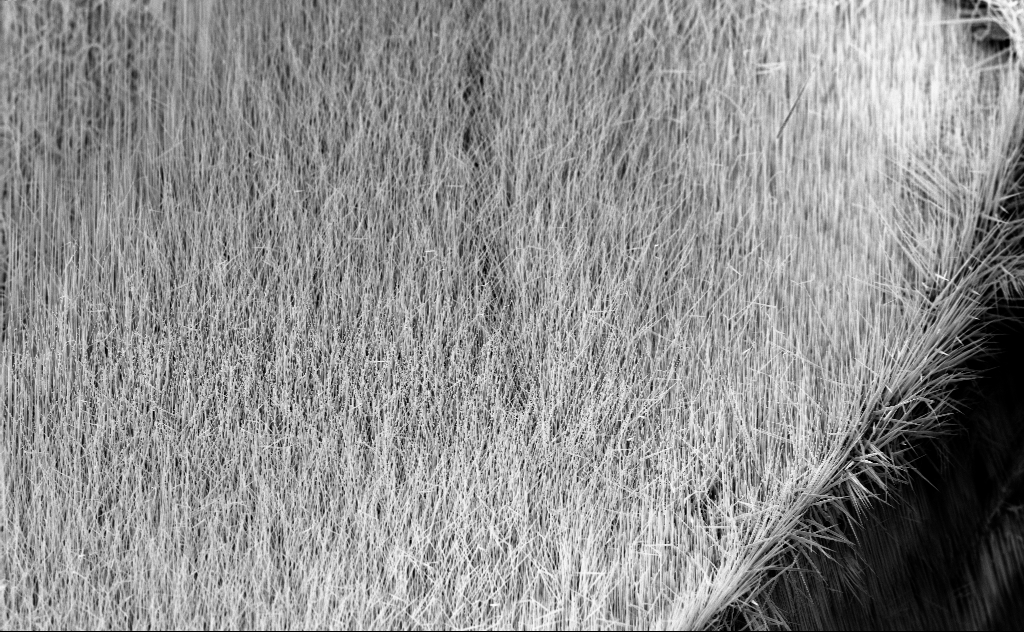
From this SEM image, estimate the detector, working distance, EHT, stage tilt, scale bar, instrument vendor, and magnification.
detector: InLens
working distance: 6 mm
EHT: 10 kV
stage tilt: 45°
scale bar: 20000 nm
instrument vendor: Zeiss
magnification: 2.2 K X